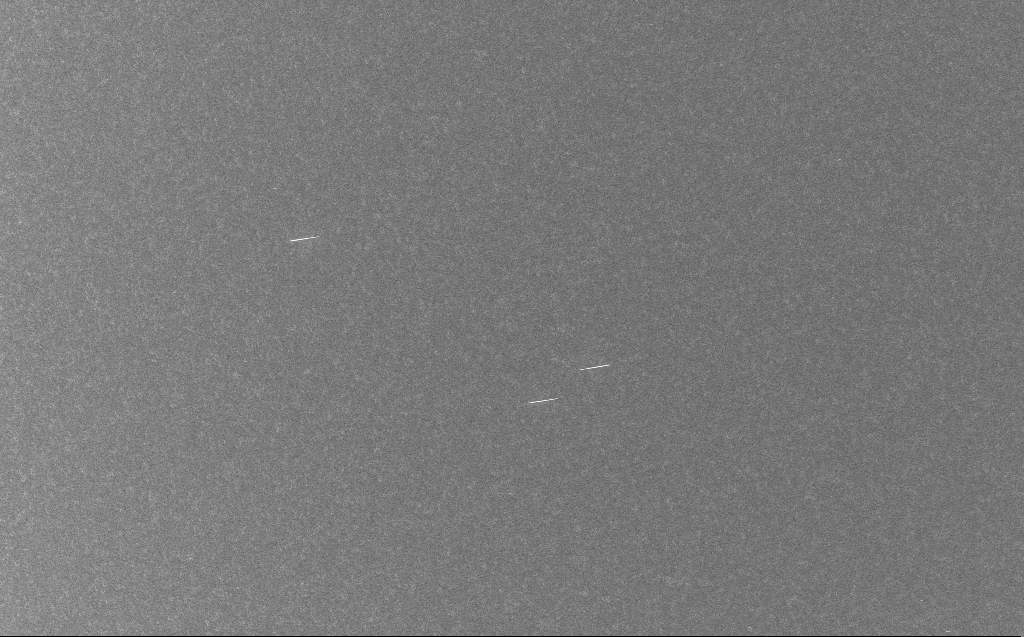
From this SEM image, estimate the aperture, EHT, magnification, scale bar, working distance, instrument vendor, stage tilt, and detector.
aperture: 30 µm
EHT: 10 kV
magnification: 2 K X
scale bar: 20000 nm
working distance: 3 mm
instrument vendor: Zeiss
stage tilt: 0°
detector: InLens